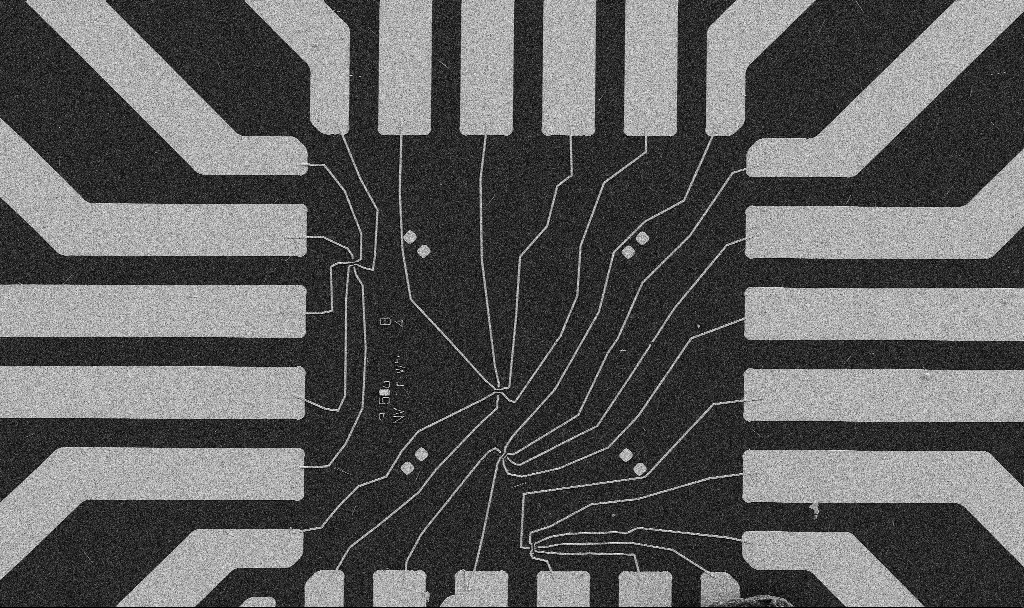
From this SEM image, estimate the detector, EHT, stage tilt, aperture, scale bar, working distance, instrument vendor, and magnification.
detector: SE2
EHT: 5 kV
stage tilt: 0°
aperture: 30 µm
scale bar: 20000 nm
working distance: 10.7 mm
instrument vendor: Zeiss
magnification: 1 K X